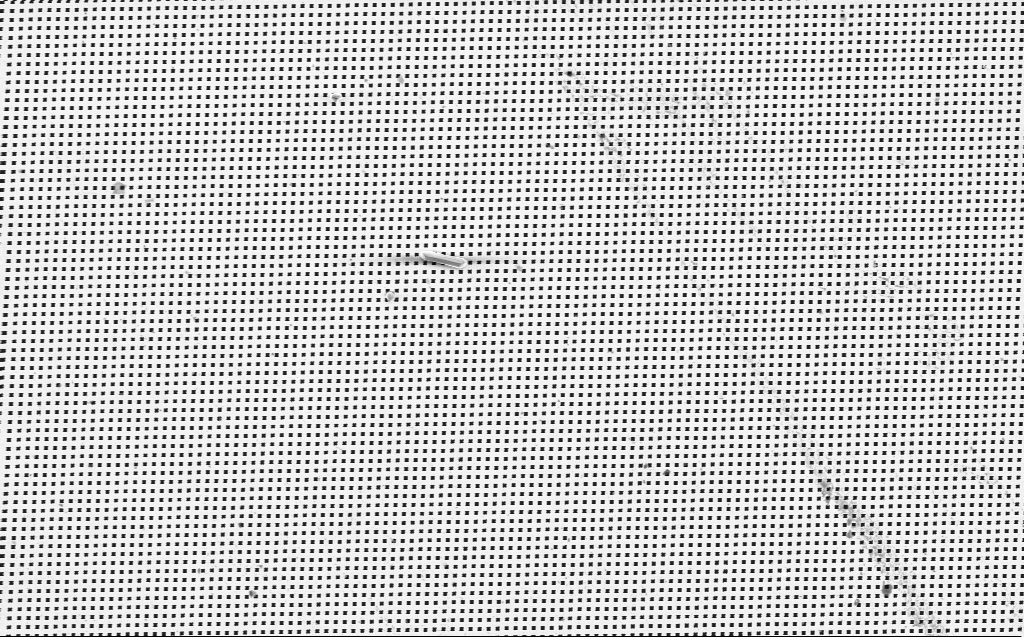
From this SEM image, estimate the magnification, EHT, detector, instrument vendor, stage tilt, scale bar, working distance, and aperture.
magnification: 6.67 K X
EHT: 3 kV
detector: InLens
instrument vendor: Zeiss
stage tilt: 0°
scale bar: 10000 nm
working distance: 6 mm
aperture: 30 µm